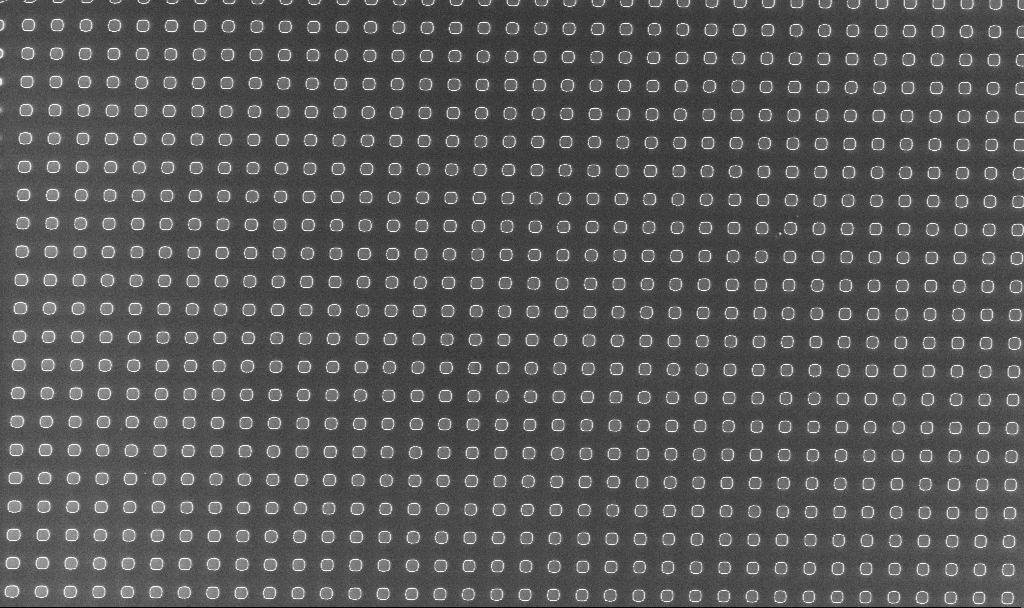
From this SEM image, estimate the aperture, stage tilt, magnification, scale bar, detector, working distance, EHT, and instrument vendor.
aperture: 30 µm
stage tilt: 0°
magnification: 1.76 K X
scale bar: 10000 nm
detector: InLens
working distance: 3.2 mm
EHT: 3 kV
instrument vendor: Zeiss